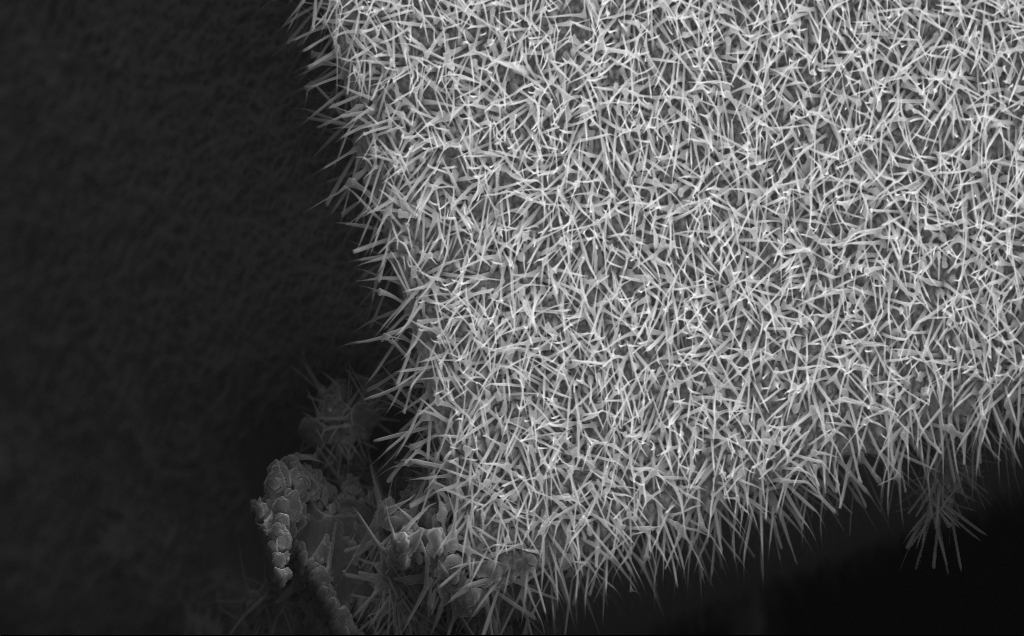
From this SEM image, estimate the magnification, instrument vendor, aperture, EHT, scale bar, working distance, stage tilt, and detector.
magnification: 6.56 K X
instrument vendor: Zeiss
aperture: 30 µm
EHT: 10 kV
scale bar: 10000 nm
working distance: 7 mm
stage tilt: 0°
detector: InLens